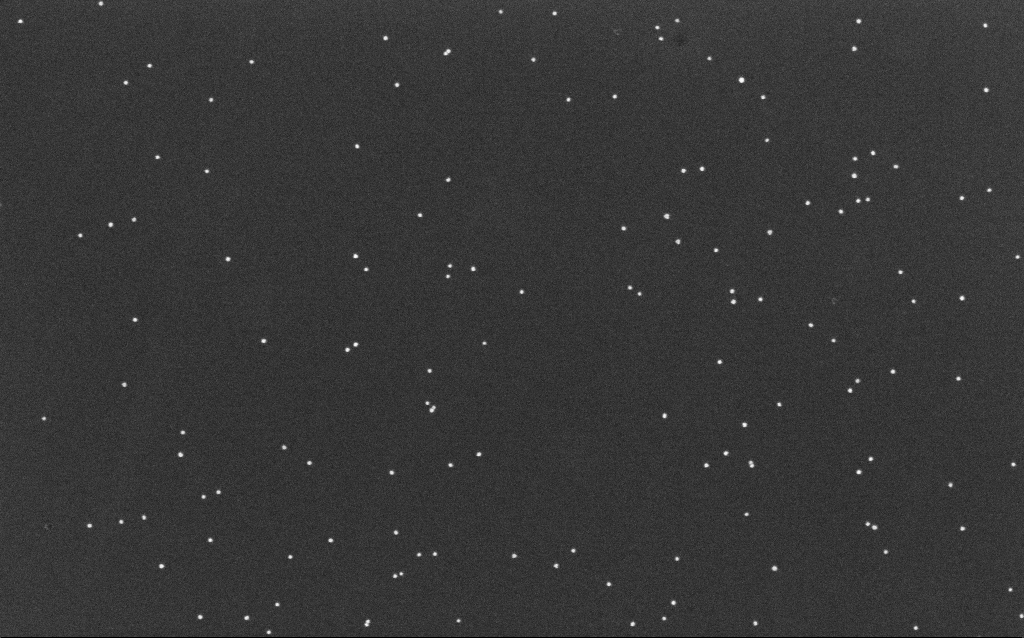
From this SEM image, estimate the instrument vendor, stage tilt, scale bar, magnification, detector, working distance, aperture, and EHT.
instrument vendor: Zeiss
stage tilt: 0°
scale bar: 200 nm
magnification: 100 K X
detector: InLens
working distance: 6.4 mm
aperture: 30 µm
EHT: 10 kV